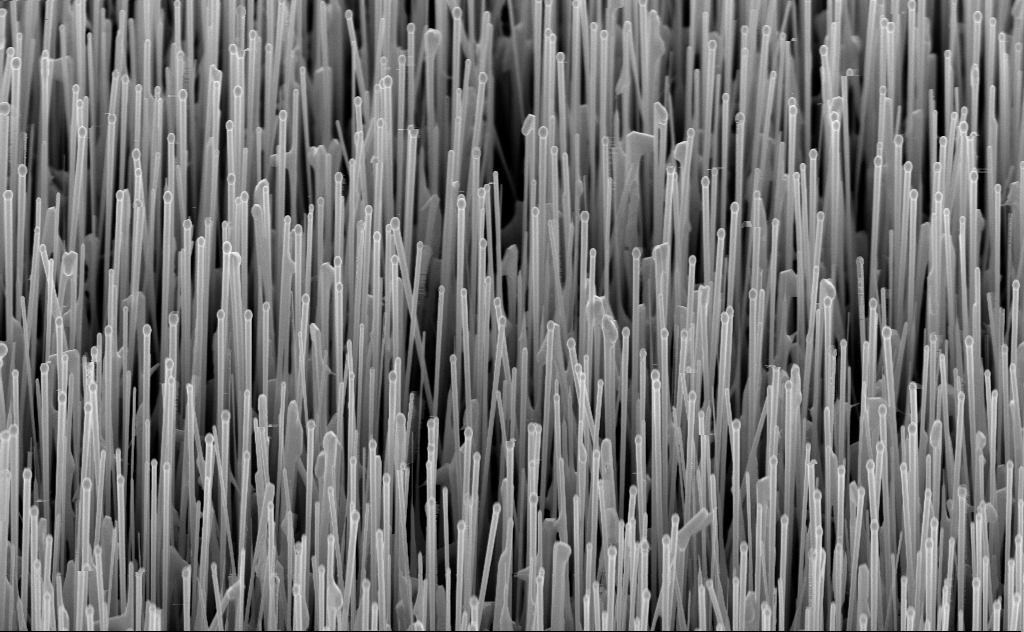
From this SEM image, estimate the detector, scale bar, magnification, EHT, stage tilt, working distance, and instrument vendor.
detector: InLens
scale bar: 2000 nm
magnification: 20 K X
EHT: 10 kV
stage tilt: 45°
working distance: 6 mm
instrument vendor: Zeiss